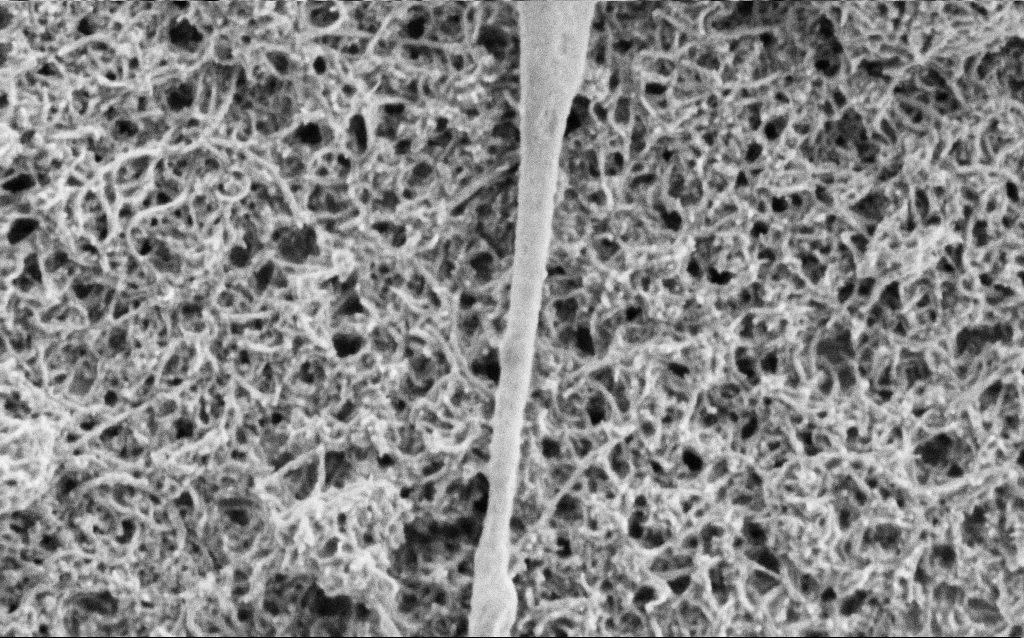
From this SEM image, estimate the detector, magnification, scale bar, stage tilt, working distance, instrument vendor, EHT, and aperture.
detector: SE2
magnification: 50 K X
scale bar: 1000 nm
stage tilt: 0°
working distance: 6.7 mm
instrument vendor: Zeiss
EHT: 1.5 kV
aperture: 30 µm